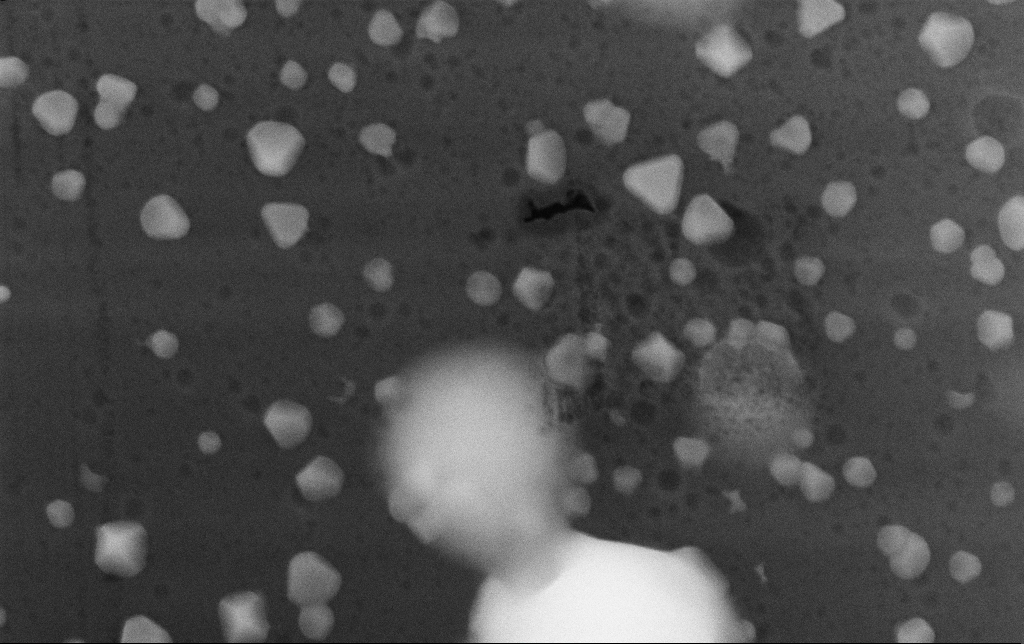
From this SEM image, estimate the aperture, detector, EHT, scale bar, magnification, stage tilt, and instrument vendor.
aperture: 30 µm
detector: InLens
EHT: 15 kV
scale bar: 200 nm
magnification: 81.81 K X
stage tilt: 0°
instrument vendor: Zeiss